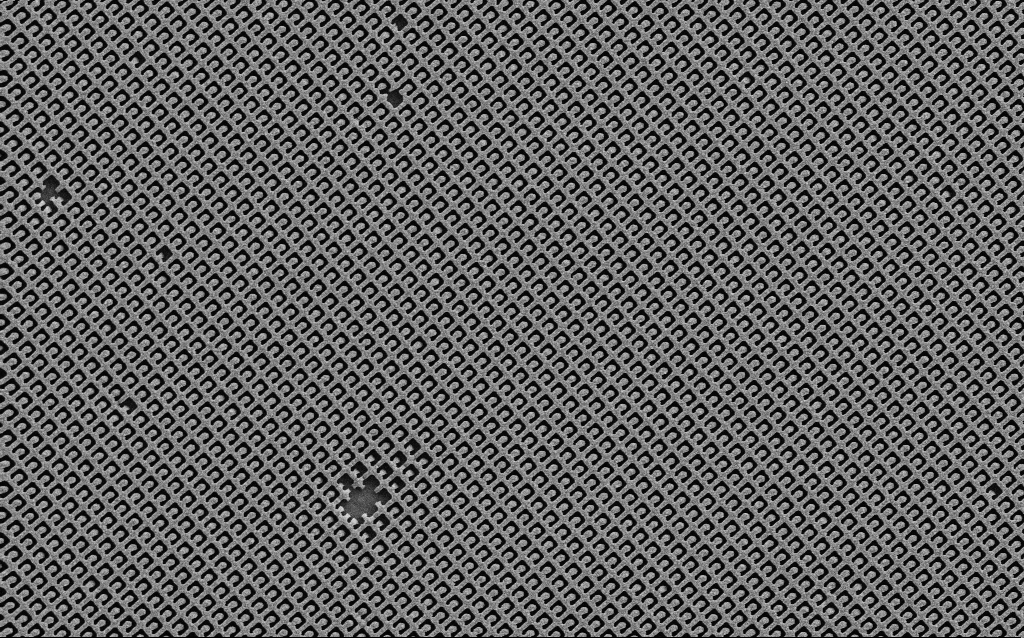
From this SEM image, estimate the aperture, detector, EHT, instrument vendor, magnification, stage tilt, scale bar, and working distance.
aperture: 30 µm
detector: SE2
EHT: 5 kV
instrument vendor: Zeiss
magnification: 14.68 K X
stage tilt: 0°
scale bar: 2000 nm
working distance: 6.1 mm